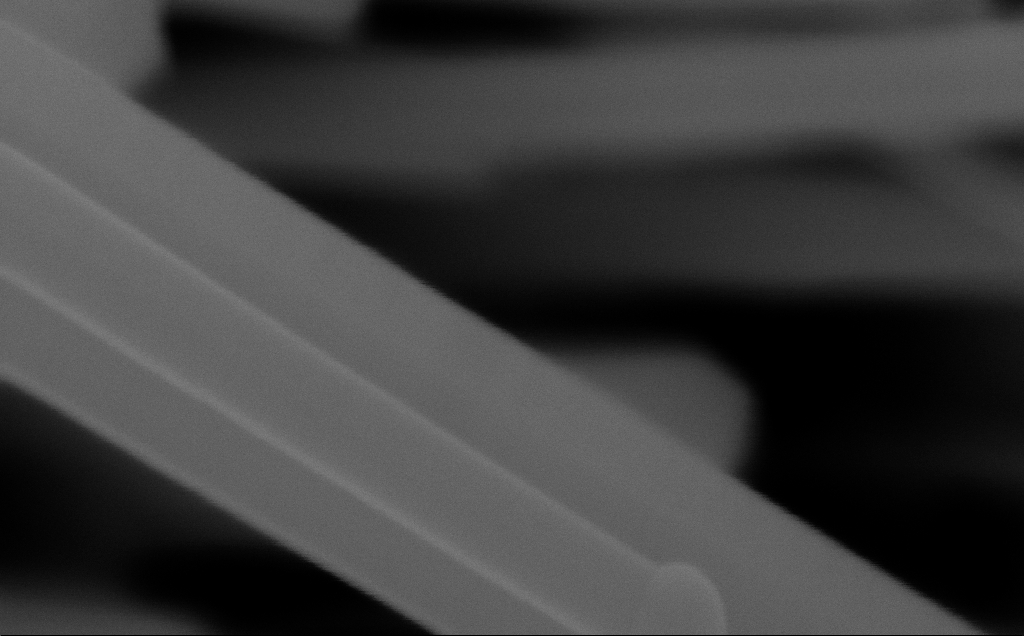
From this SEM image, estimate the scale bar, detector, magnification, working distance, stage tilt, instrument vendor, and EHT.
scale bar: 100 nm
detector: InLens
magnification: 449.92 K X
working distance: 6 mm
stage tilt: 0°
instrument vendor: Zeiss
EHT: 10 kV